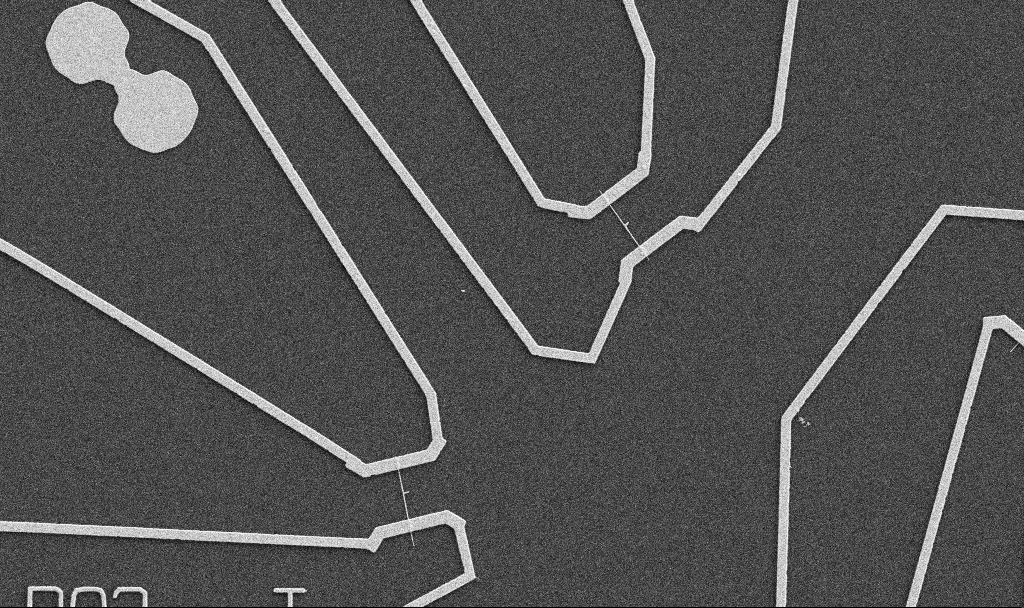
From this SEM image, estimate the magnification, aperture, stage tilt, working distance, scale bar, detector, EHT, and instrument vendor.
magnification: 5 K X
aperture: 30 µm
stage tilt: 0°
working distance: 10.7 mm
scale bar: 10000 nm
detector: SE2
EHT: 5 kV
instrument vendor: Zeiss